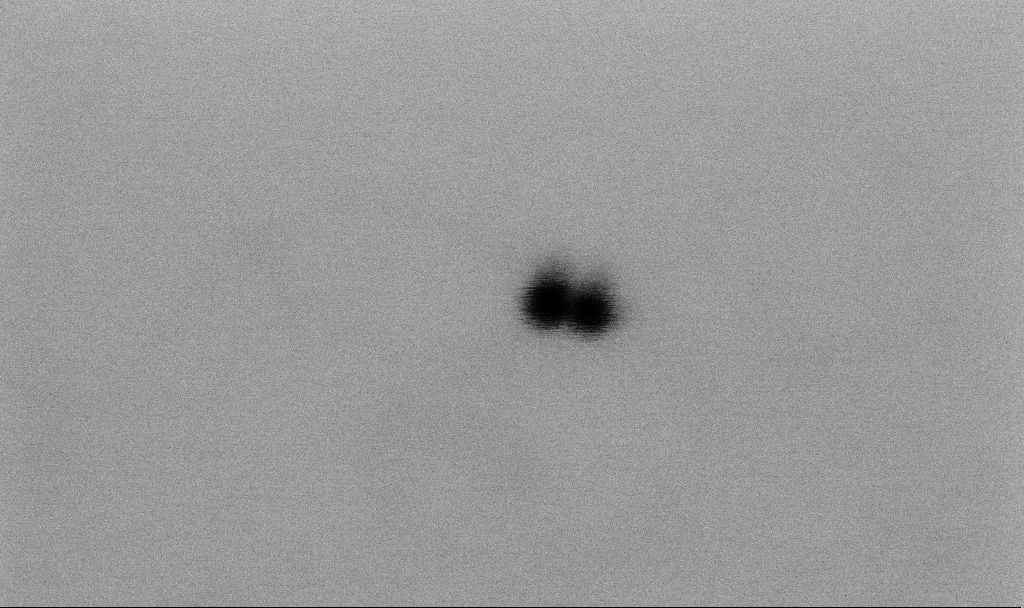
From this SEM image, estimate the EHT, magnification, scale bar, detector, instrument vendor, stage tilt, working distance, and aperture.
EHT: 2 kV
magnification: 748 K X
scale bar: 20 nm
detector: SE2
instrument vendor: Zeiss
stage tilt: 0°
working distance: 5.5 mm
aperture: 30 µm